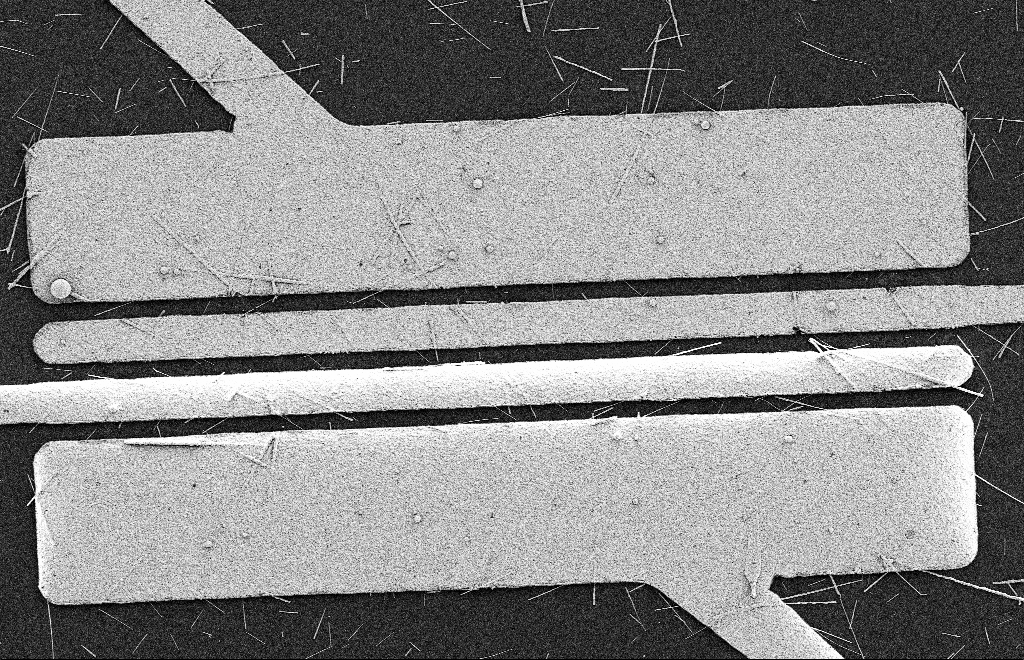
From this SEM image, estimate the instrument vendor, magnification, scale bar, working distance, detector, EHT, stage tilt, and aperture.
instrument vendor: Zeiss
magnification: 5.62 K X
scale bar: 2000 nm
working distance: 9 mm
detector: SE2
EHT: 2 kV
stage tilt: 0°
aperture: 20 µm